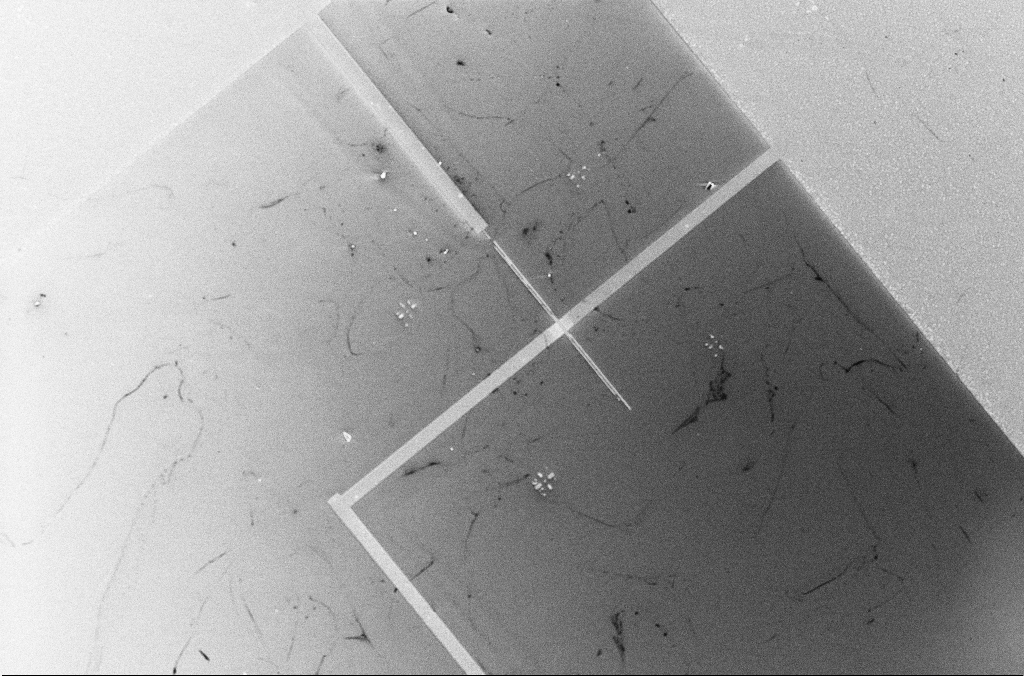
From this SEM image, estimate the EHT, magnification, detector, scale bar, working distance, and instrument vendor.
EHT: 5 kV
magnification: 1.02 K X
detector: InLens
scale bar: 20000 nm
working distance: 3.4 mm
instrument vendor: Zeiss